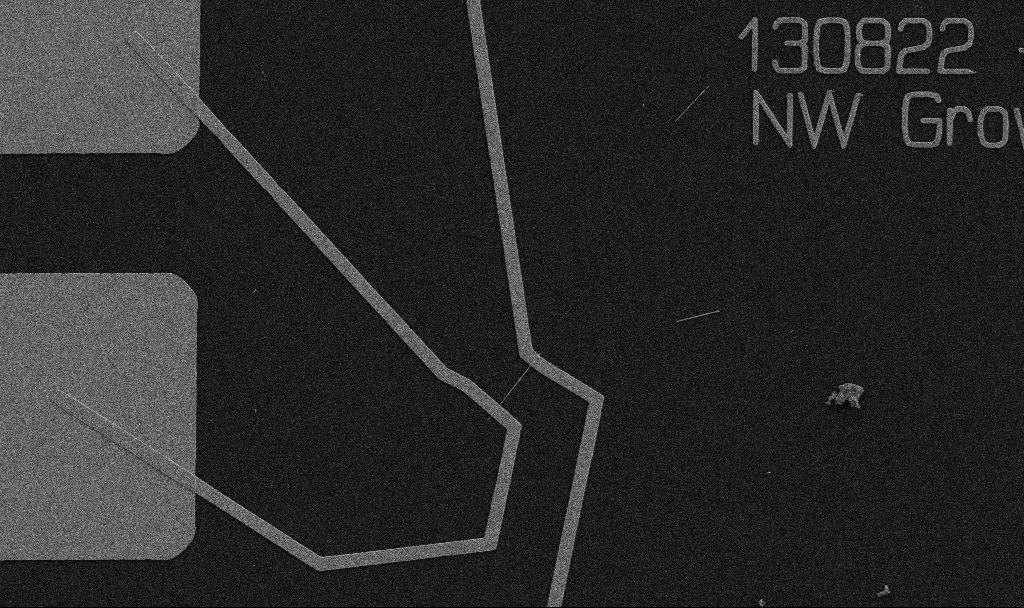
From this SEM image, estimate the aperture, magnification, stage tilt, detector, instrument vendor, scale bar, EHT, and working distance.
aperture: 30 µm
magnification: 5 K X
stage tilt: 0°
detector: SE2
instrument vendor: Zeiss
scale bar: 10000 nm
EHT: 5 kV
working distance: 10.7 mm